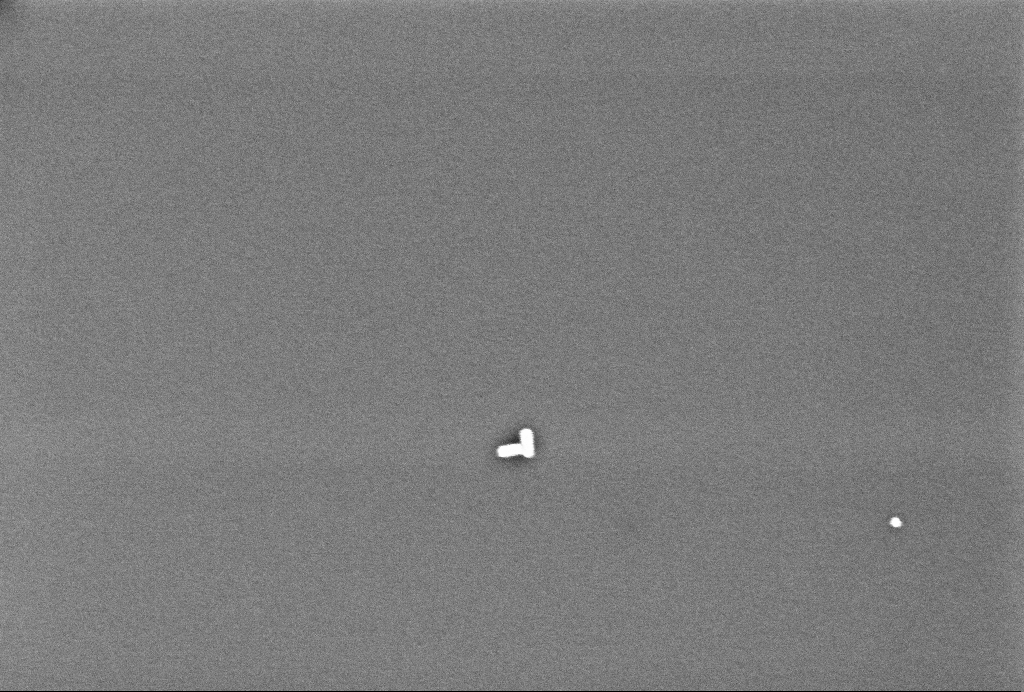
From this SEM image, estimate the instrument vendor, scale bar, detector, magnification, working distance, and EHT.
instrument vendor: Zeiss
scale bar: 200 nm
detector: InLens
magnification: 134.35 K X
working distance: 3.3 mm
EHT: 2 kV